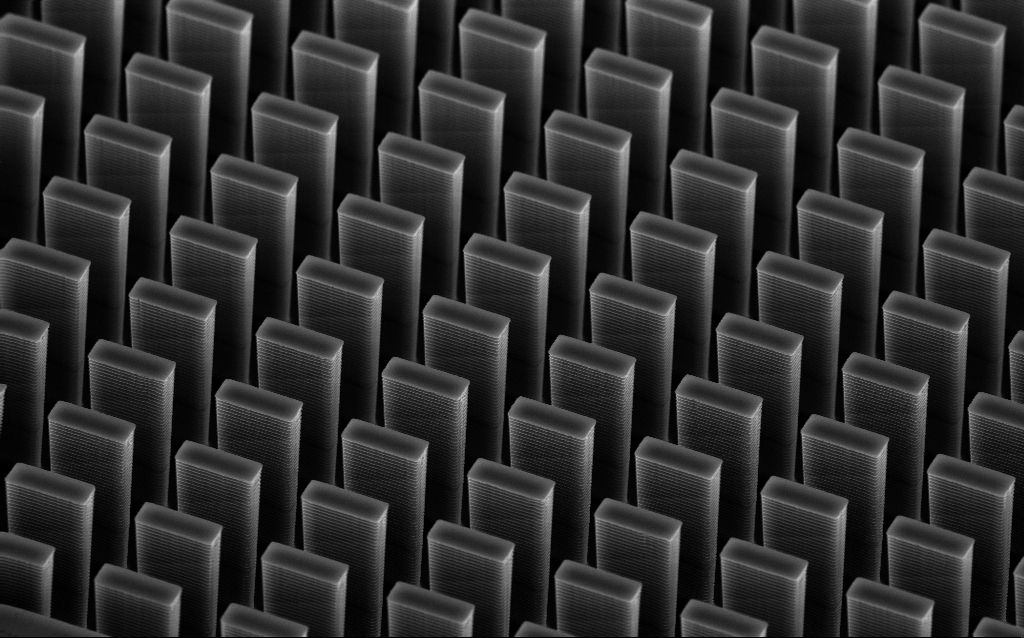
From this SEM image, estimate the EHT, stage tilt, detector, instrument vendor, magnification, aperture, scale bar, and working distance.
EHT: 10 kV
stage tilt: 45°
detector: InLens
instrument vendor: Zeiss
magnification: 6.42 K X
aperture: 30 µm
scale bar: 10000 nm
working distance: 5.2 mm